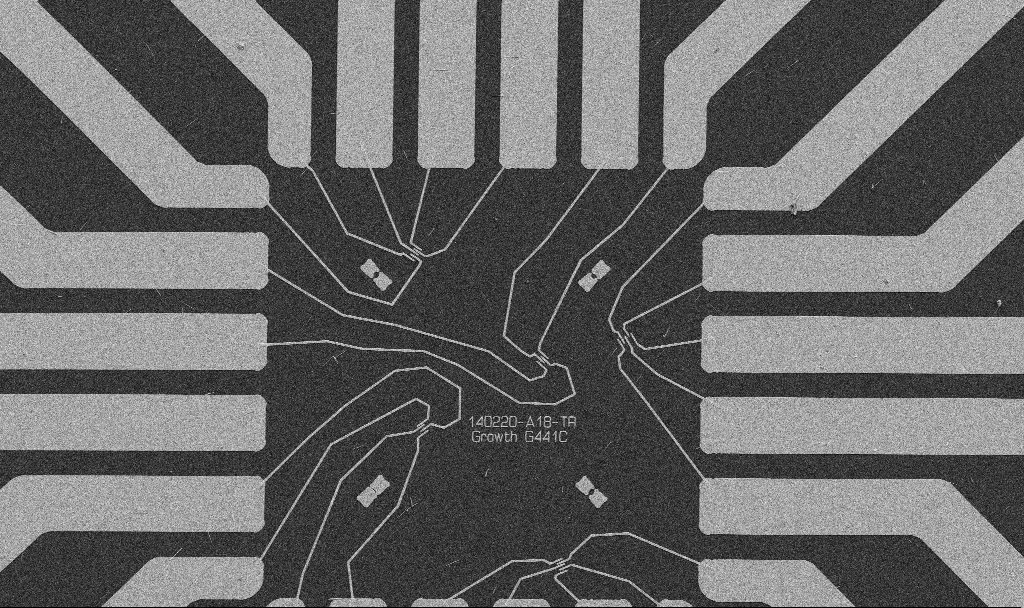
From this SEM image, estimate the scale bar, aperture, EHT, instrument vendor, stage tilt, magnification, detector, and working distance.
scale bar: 20000 nm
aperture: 30 µm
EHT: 5 kV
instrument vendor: Zeiss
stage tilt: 0°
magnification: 1 K X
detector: SE2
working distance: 10.7 mm